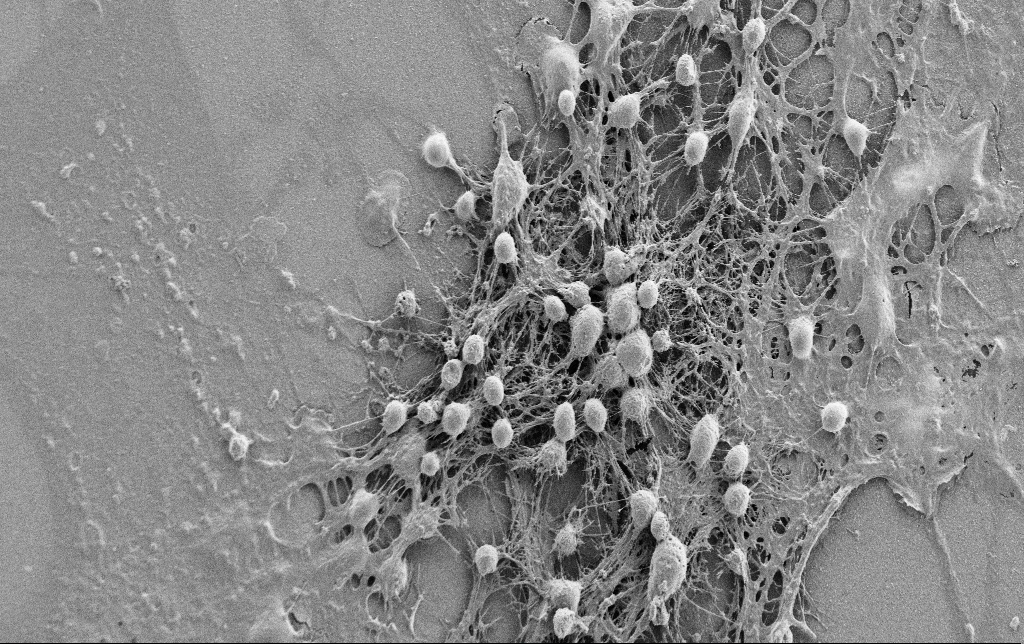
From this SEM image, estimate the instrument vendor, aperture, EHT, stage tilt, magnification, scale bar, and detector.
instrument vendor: Zeiss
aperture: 30 µm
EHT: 0.9 kV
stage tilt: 0°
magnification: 2 K X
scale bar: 20000 nm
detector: SE2